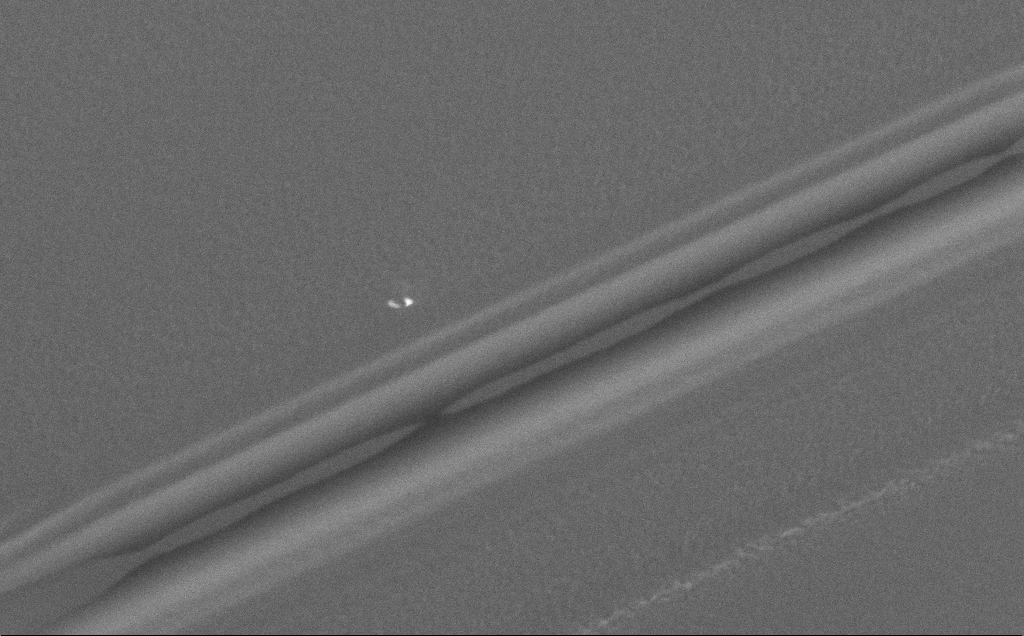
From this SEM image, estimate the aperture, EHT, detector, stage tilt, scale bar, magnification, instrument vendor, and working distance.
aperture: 30 µm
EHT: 1 kV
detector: SE2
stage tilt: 0°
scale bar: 10000 nm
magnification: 3.54 K X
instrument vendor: Zeiss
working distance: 6 mm